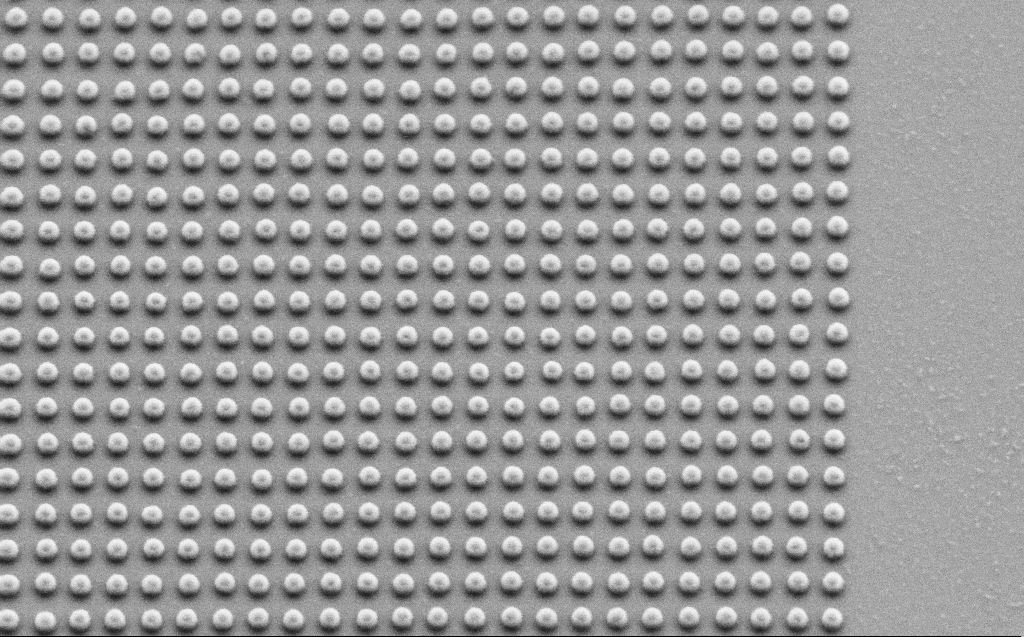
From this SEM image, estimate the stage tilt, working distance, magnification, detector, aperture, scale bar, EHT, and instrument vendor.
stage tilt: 30°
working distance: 5 mm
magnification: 33.07 K X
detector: SE2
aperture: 30 µm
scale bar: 1000 nm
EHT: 2.5 kV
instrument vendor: Zeiss